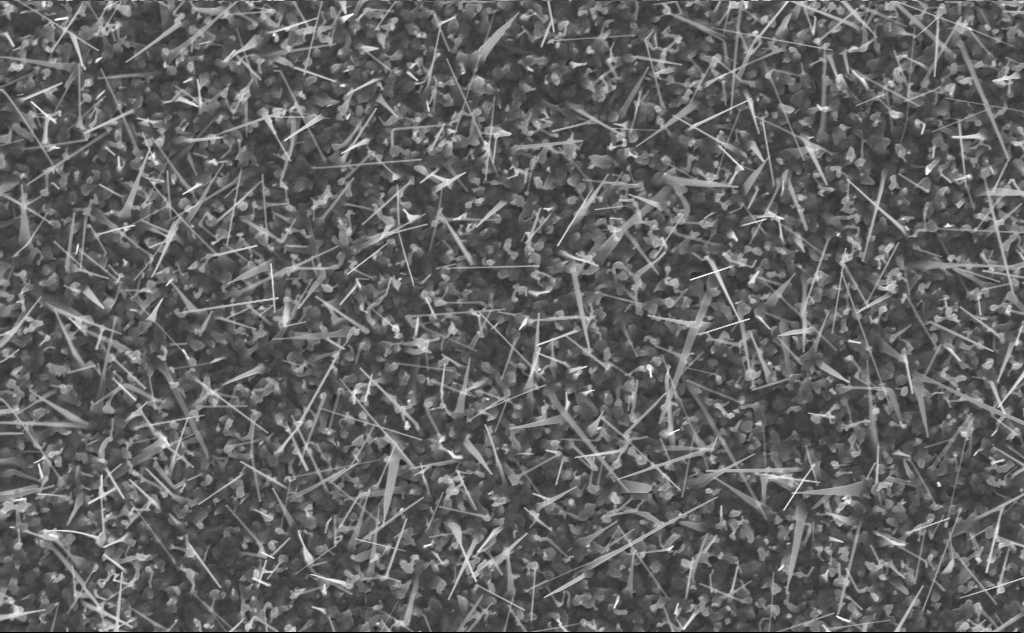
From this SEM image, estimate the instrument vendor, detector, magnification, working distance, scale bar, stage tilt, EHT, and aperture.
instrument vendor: Zeiss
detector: InLens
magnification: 20 K X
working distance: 5 mm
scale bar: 2000 nm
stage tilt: -0°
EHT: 10 kV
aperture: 30 µm